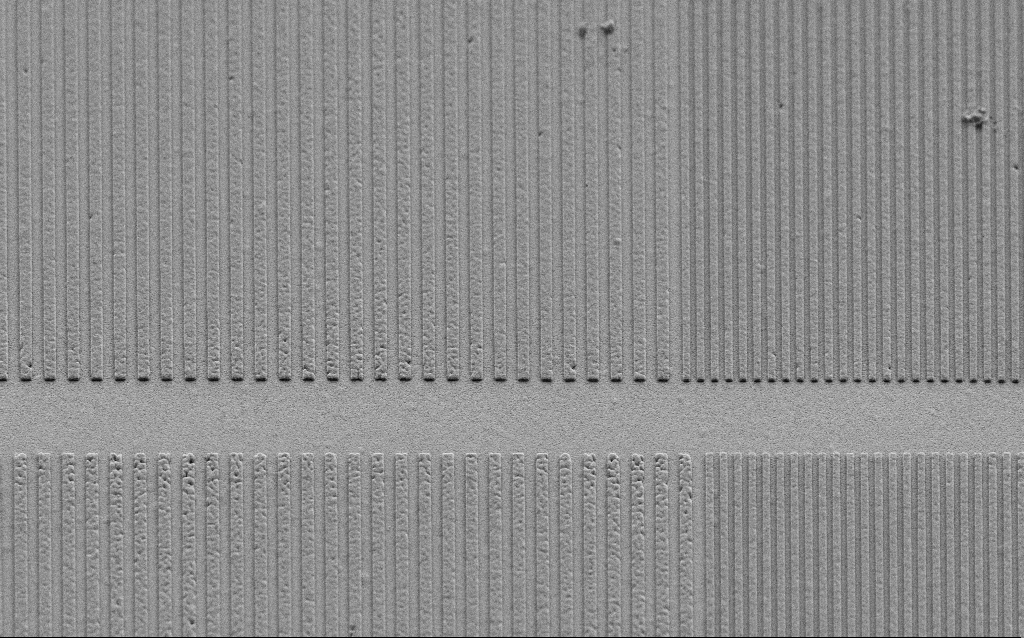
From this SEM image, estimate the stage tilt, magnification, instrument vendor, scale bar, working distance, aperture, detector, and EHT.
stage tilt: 45°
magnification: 8.64 K X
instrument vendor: Zeiss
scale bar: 2000 nm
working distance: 6.6 mm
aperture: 30 µm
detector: SE2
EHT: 3 kV